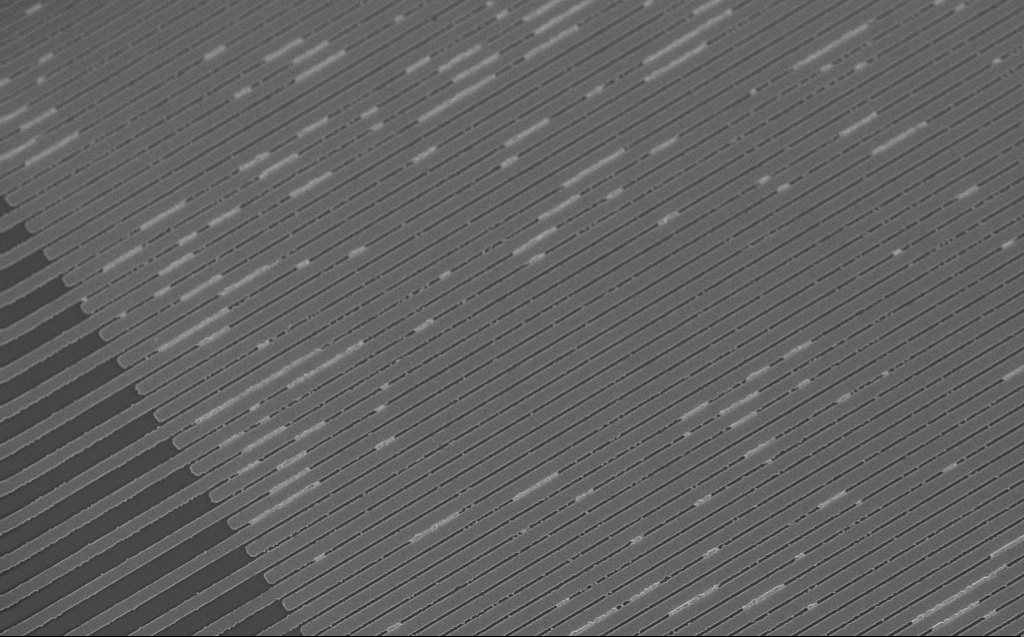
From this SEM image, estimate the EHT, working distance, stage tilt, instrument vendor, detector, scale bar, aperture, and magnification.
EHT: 3 kV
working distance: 4 mm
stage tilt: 20°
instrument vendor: Zeiss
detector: InLens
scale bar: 20000 nm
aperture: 30 µm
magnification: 1.6 K X